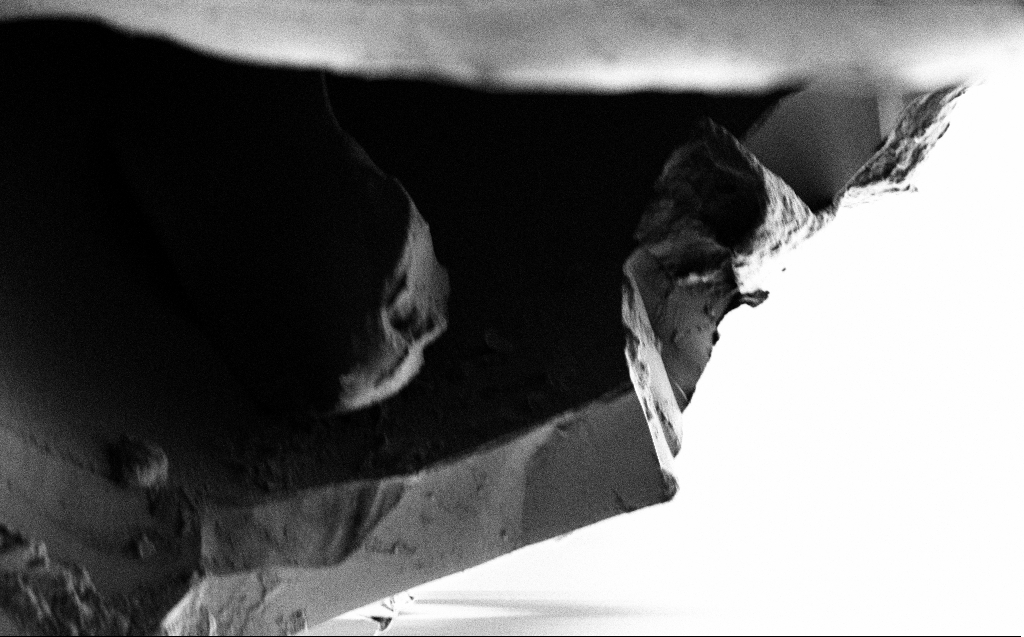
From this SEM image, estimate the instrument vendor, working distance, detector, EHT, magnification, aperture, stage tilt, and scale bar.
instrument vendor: Zeiss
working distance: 3 mm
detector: SE2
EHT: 1 kV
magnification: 7.47 K X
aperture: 30 µm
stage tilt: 45°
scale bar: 2000 nm